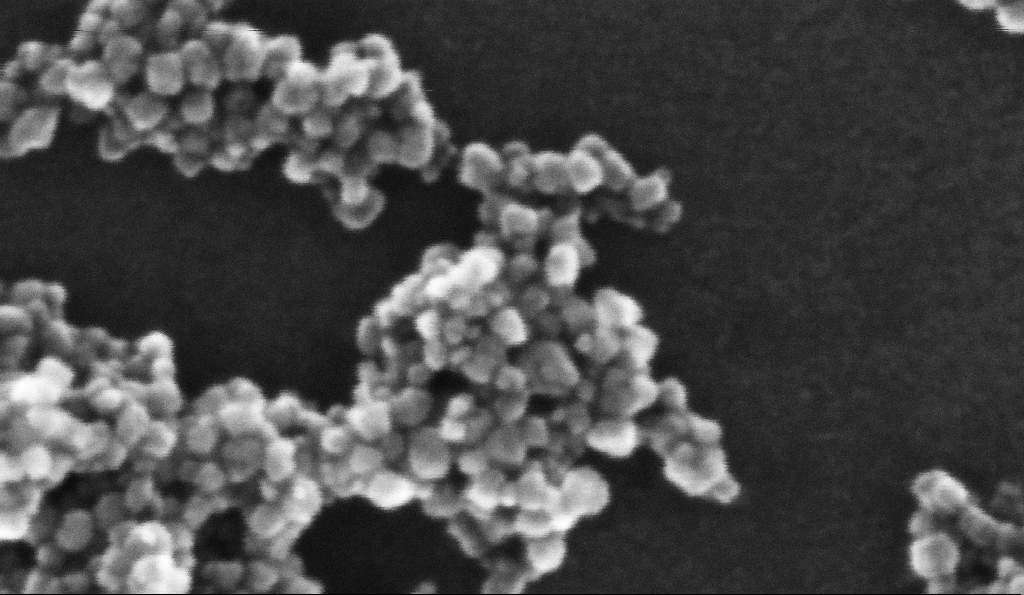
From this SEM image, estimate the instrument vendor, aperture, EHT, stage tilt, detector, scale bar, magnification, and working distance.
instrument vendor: Zeiss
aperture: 30 µm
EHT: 10 kV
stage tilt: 0°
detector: InLens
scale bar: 20 nm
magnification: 849.63 K X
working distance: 5.2 mm